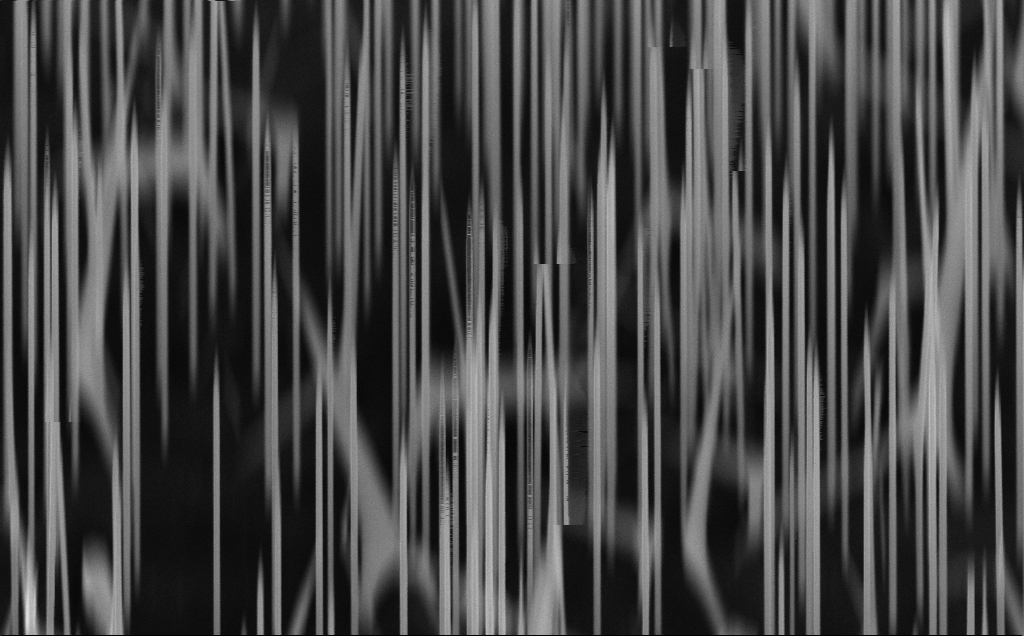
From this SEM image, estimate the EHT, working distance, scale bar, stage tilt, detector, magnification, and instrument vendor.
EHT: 10 kV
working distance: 9 mm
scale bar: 1000 nm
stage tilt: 45°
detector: InLens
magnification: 43.9 K X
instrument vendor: Zeiss